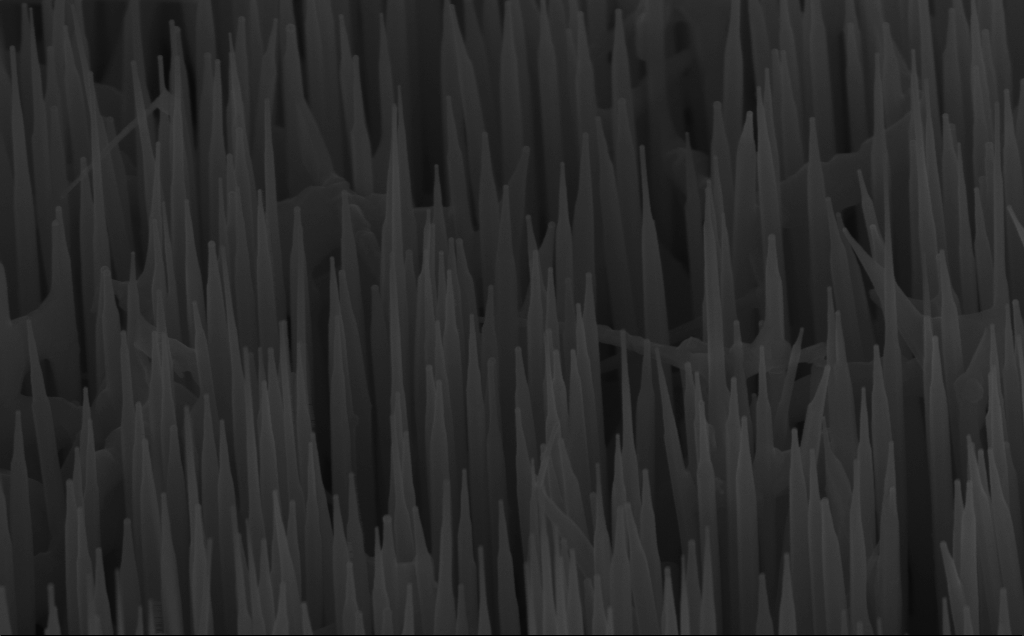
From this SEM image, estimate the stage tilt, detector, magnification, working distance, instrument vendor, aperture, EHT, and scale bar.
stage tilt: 45°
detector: InLens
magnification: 80 K X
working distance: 6 mm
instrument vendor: Zeiss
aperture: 30 µm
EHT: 10 kV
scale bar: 200 nm